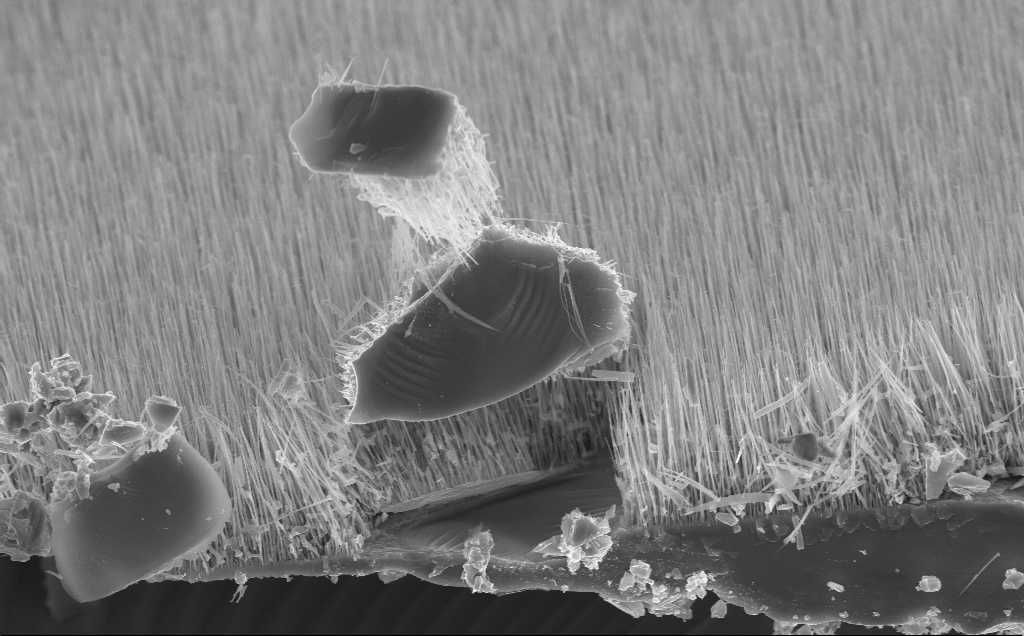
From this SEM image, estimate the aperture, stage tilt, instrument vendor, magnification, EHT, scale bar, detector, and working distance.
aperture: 30 µm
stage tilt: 45°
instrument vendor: Zeiss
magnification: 11.42 K X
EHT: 10 kV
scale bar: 2000 nm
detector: InLens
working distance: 7 mm